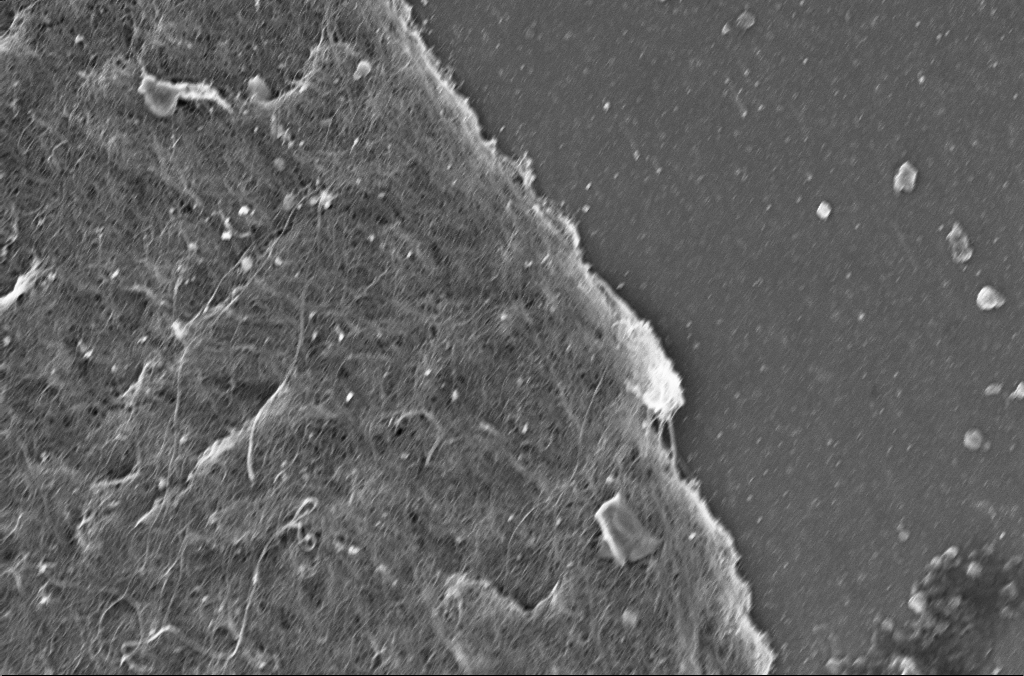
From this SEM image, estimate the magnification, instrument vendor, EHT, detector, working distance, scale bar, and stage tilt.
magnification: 10 K X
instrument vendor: Zeiss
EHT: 5 kV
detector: InLens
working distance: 2.9 mm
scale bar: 2000 nm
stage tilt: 0°